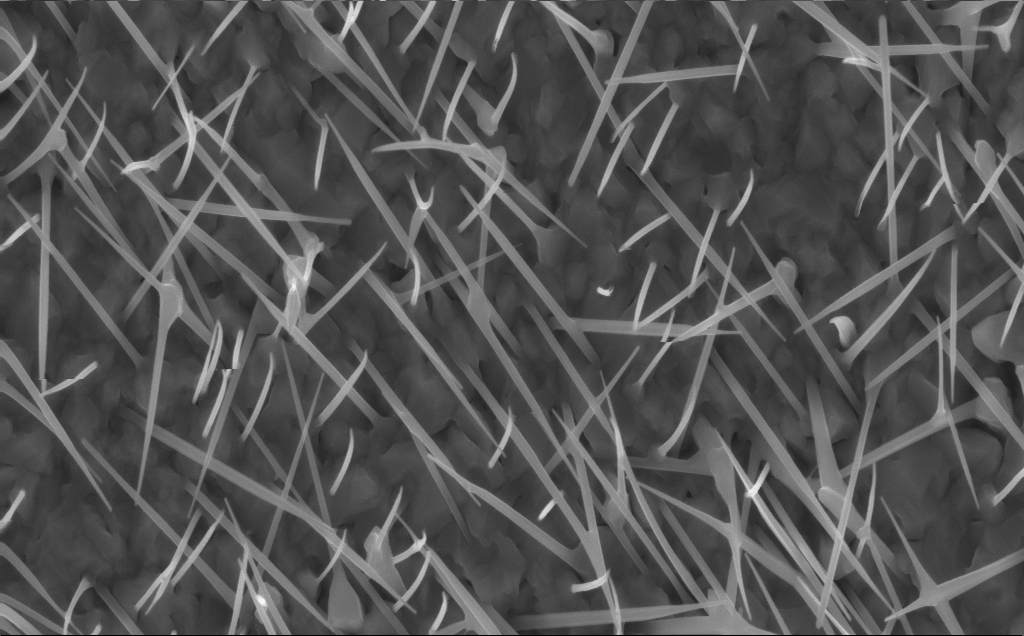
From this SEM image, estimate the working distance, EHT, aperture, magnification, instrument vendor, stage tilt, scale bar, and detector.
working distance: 5 mm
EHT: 10 kV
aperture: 30 µm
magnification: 40 K X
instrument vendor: Zeiss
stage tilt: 0°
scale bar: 1000 nm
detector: InLens